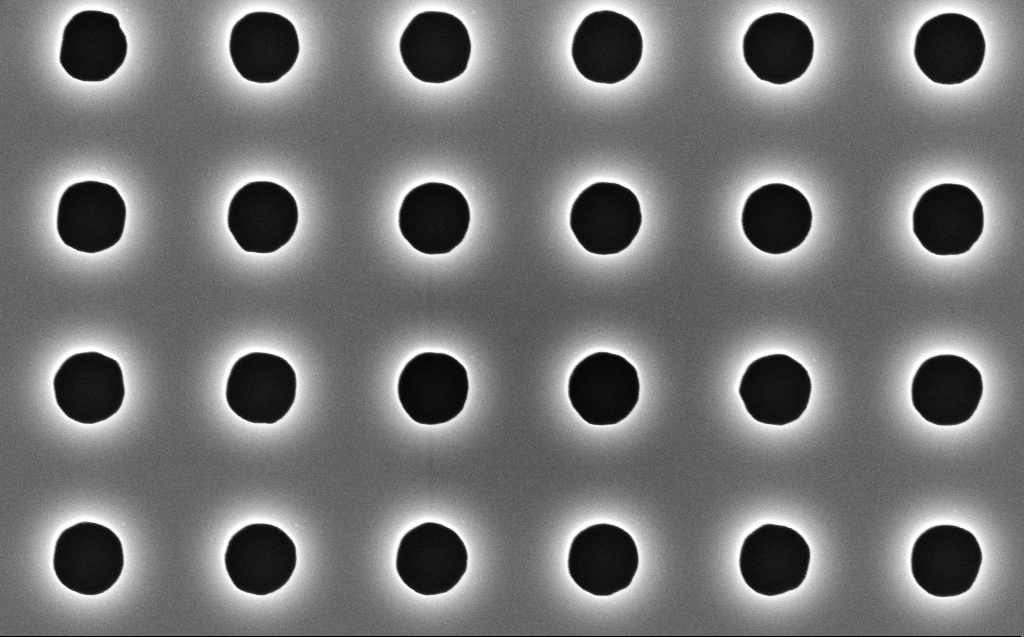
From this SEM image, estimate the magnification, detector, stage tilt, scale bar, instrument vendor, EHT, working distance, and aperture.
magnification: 60 K X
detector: InLens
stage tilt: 0°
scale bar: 1000 nm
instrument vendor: Zeiss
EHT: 5 kV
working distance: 7 mm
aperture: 30 µm